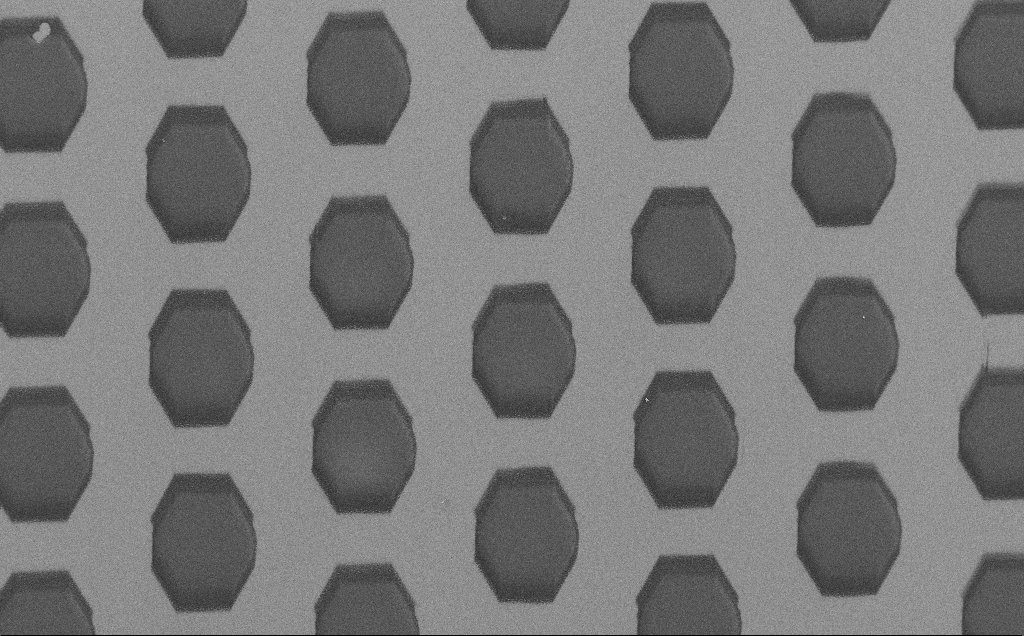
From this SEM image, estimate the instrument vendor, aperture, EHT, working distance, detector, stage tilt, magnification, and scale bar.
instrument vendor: Zeiss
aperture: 30 µm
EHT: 1.5 kV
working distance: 6 mm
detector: SE2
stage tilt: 0°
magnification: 0.682 K X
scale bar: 100000 nm